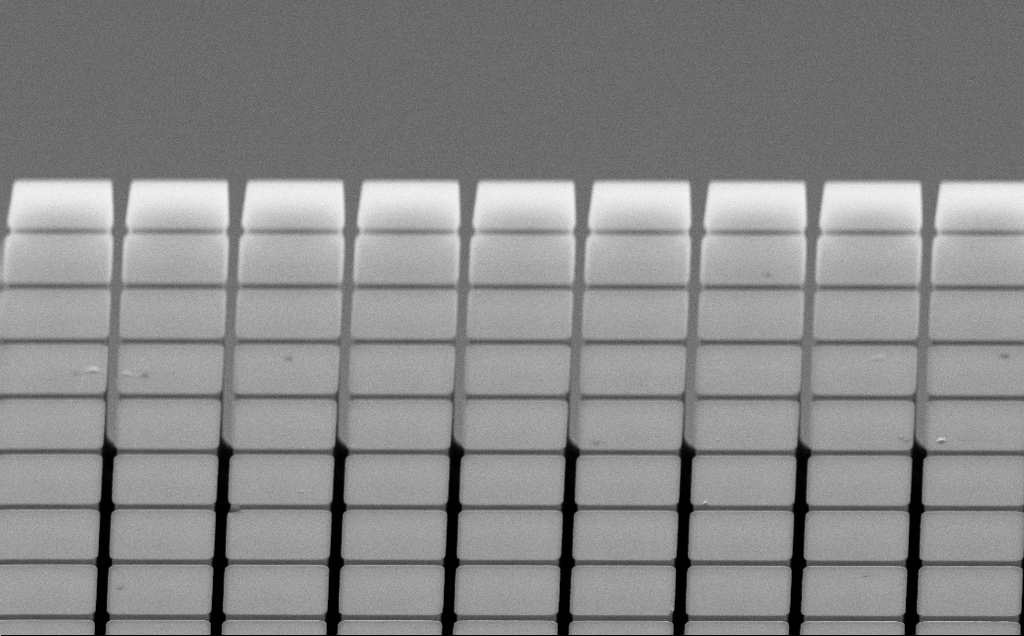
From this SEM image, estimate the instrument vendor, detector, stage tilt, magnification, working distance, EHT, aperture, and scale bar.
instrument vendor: Zeiss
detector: SE2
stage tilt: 60°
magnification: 8.45 K X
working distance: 9 mm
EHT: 20 kV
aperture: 30 µm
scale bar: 2000 nm